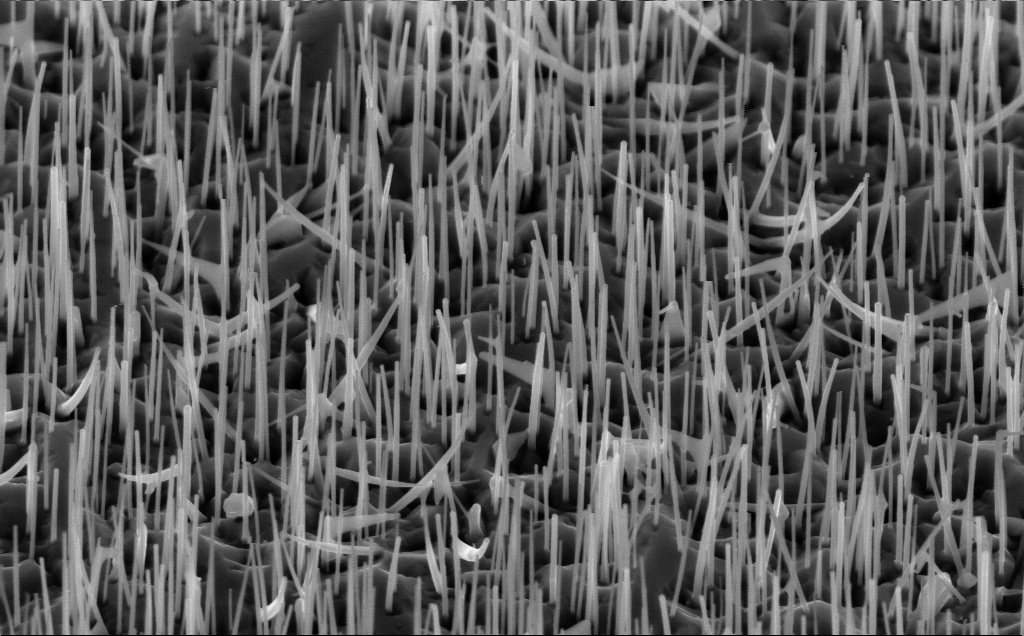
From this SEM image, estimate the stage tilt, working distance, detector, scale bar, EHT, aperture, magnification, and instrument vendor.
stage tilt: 45°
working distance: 5 mm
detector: InLens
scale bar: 1000 nm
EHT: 10 kV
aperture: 30 µm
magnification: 40 K X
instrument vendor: Zeiss